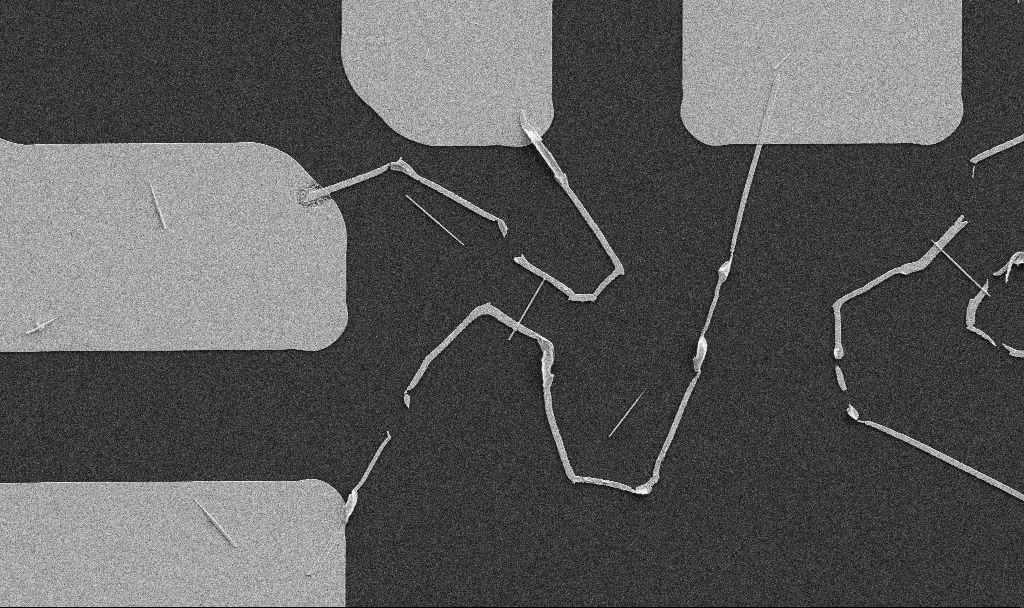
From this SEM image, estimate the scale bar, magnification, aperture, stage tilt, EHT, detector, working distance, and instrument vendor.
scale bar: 10000 nm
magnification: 5 K X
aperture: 30 µm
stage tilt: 0°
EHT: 5 kV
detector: SE2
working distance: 10.7 mm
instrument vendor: Zeiss